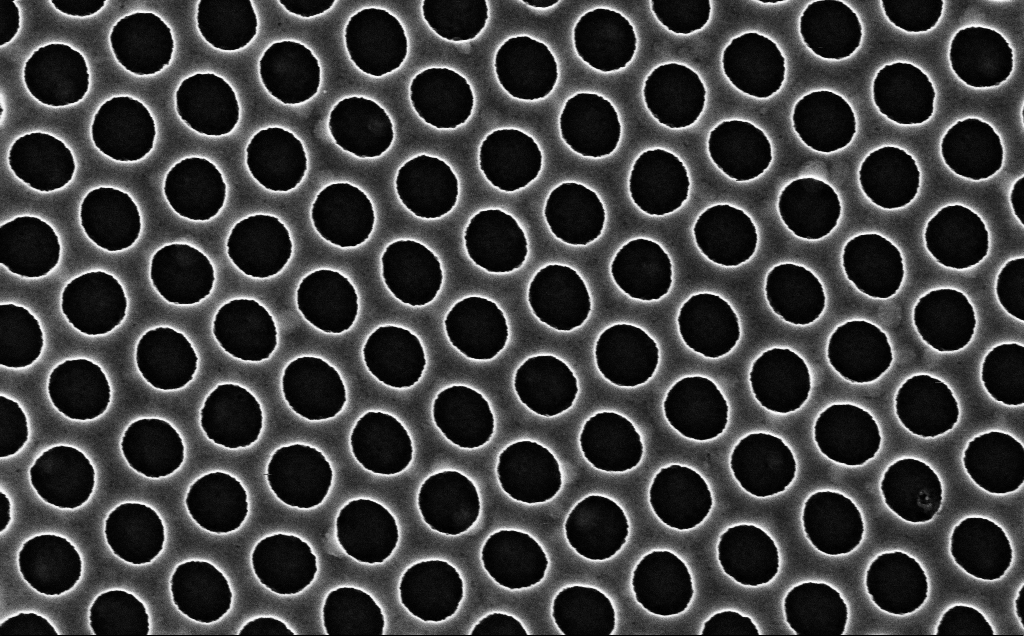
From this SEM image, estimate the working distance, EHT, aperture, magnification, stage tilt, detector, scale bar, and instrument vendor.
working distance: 2.4 mm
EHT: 3 kV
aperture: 30 µm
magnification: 90 K X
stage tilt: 0°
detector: InLens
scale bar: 200 nm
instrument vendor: Zeiss